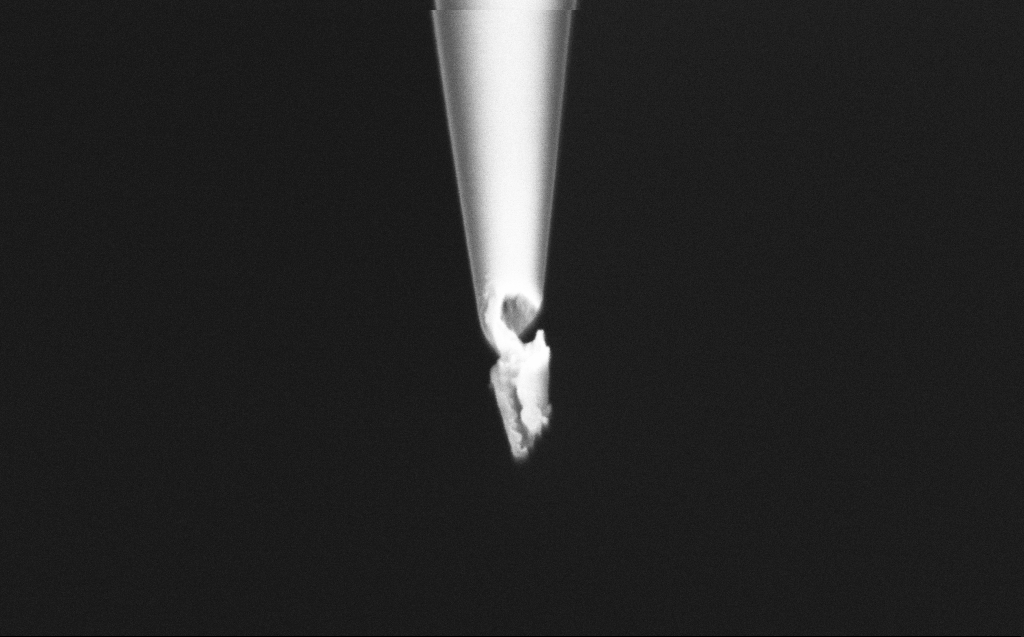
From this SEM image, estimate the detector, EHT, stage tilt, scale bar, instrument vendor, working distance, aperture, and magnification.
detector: InLens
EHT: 2 kV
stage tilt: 45°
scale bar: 200 nm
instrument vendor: Zeiss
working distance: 6 mm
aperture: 30 µm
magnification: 100 K X